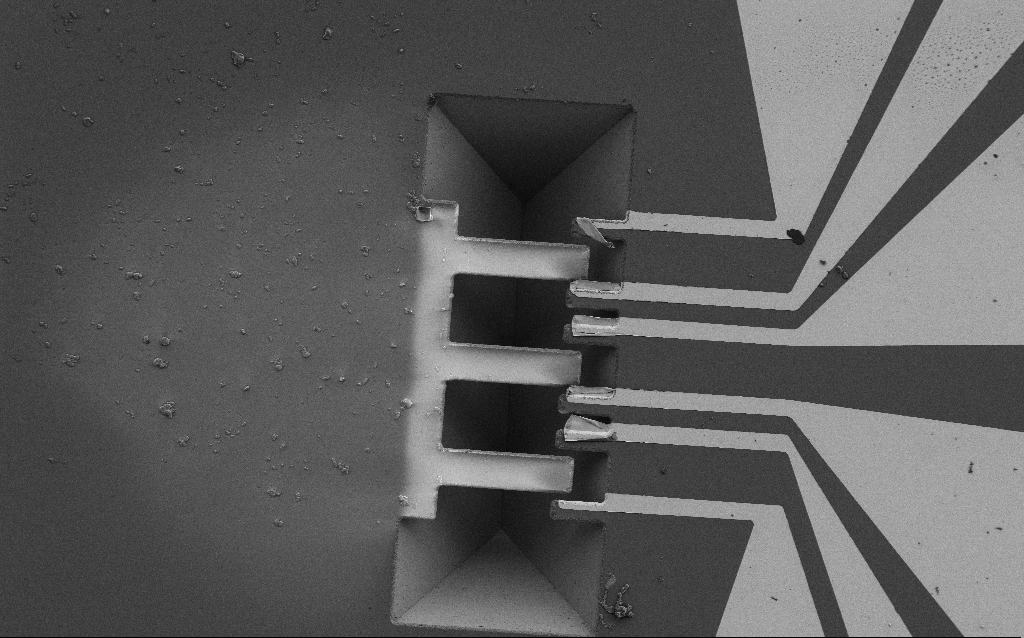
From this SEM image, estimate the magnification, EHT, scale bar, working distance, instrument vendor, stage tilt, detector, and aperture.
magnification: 0.65 K X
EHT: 2 kV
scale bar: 100000 nm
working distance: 9.7 mm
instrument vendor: Zeiss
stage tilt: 0.1°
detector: SE2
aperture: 30 µm